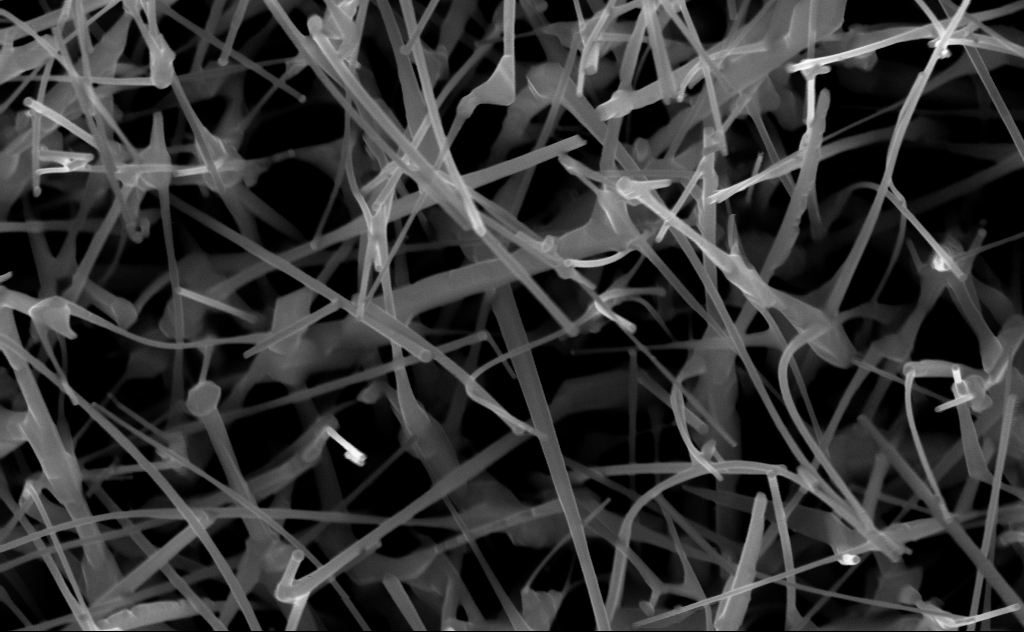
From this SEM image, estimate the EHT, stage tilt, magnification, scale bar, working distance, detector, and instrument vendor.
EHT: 10 kV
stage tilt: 0°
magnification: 80 K X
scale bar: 200 nm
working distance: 6 mm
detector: InLens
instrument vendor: Zeiss